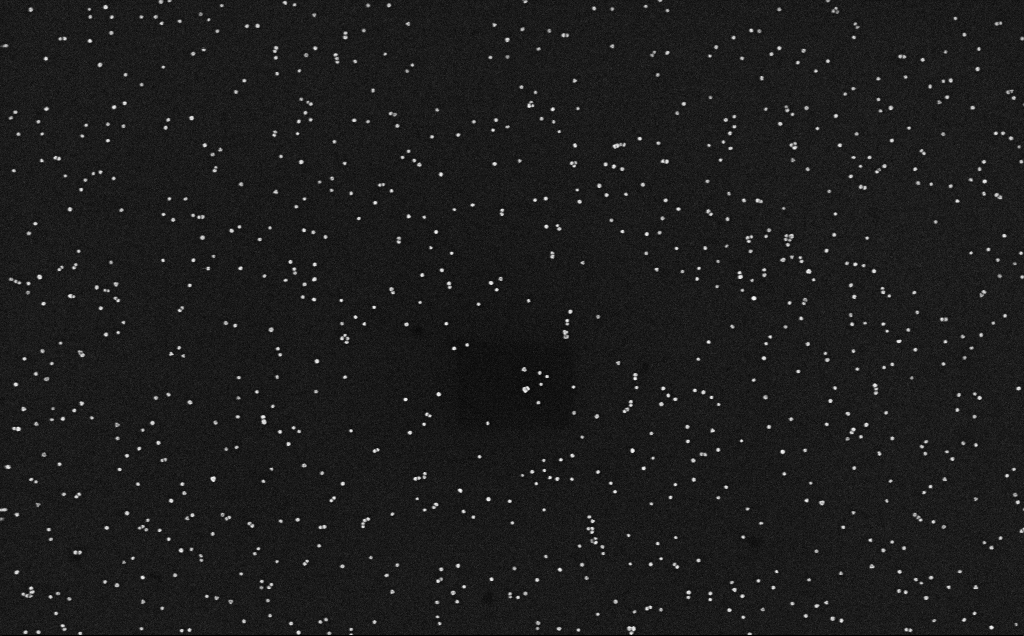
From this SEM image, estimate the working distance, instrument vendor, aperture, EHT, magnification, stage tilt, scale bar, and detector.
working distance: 3.2 mm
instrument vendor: Zeiss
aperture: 30 µm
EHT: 10 kV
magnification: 100 K X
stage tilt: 0°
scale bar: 200 nm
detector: InLens